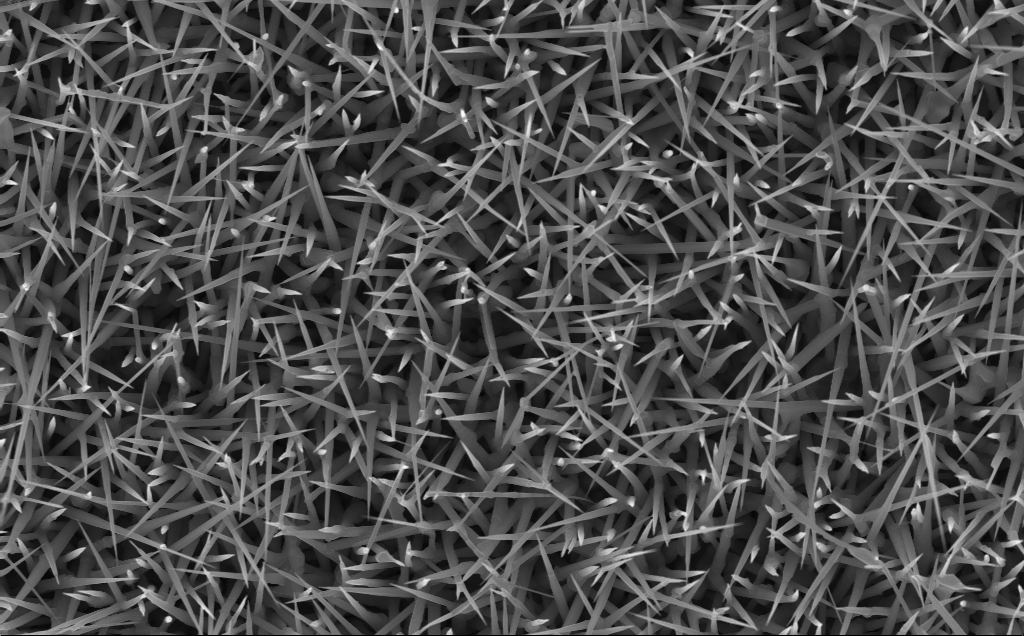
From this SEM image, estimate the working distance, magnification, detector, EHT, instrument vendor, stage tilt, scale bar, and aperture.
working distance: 5 mm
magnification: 20 K X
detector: InLens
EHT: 10 kV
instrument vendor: Zeiss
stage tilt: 0°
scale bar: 2000 nm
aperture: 30 µm